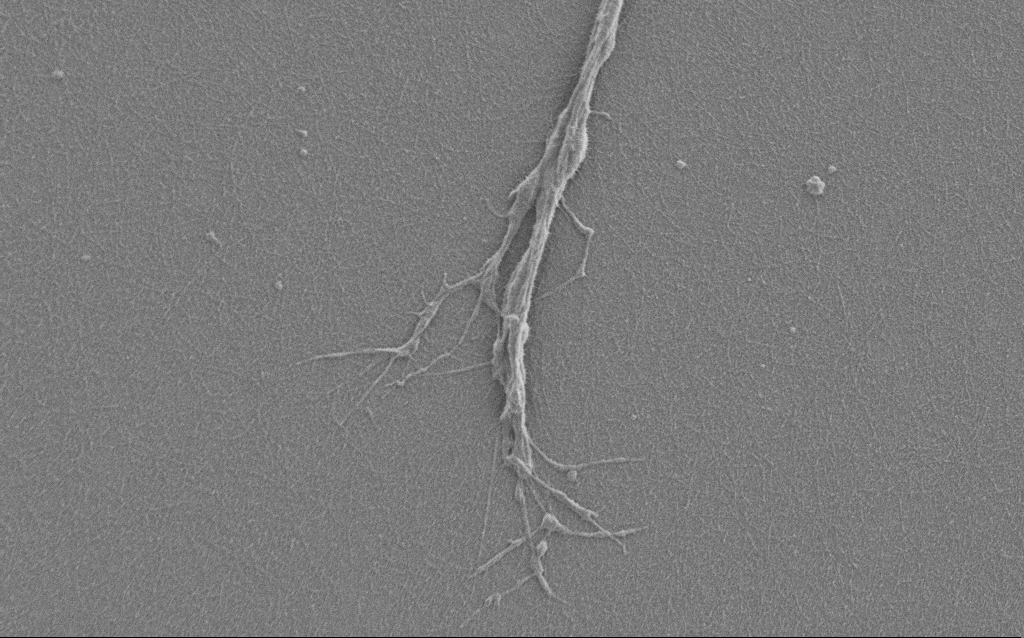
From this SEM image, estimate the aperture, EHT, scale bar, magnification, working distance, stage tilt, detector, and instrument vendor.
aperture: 30 µm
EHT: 1 kV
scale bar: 2000 nm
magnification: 7.5 K X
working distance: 6 mm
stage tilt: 0°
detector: SE2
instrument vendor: Zeiss